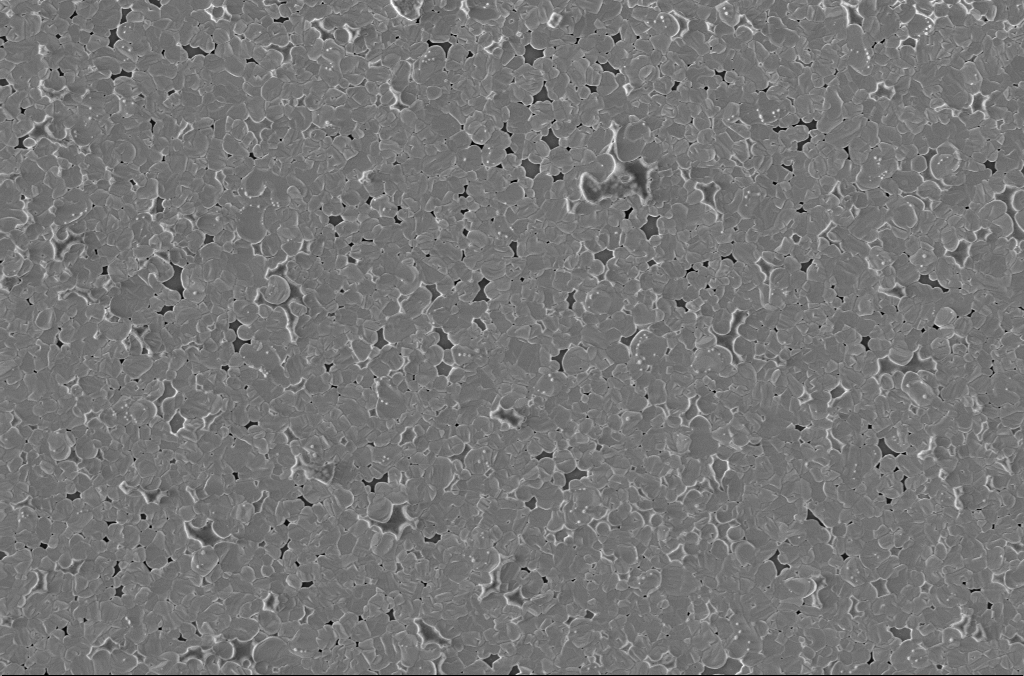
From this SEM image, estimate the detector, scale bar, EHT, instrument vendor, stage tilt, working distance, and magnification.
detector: InLens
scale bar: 2000 nm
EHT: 2 kV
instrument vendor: Zeiss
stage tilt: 0°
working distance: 3 mm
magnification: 10 K X